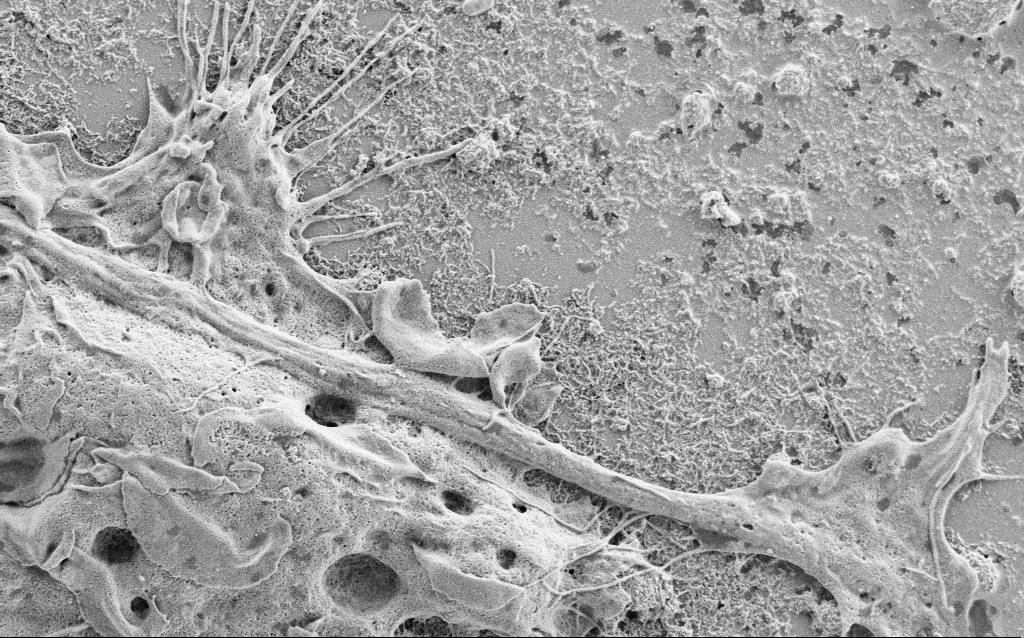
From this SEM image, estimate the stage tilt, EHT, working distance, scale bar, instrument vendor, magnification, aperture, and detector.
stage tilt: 0°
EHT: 1.5 kV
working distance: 6.8 mm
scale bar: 2000 nm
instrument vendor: Zeiss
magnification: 10 K X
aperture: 30 µm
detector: SE2